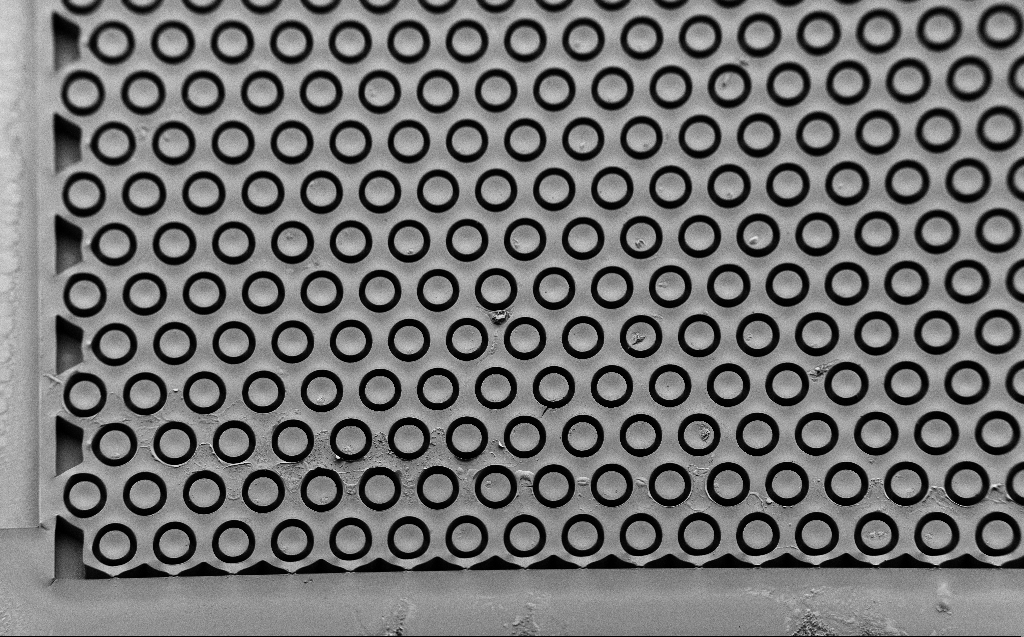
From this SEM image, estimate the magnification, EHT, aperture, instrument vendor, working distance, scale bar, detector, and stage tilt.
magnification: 0.268 K X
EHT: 2 kV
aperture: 30 µm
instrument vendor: Zeiss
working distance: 7 mm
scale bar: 100000 nm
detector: SE2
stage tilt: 45°